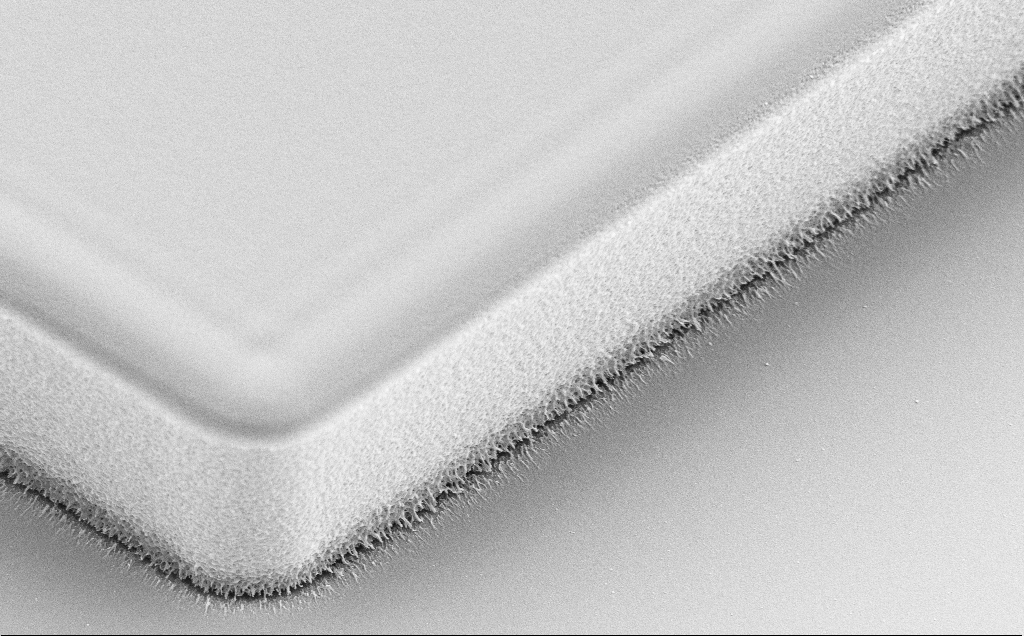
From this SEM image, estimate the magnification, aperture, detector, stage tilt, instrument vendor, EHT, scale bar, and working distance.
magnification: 13.54 K X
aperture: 30 µm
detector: SE2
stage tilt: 30°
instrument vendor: Zeiss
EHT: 5 kV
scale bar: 1000 nm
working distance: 8 mm